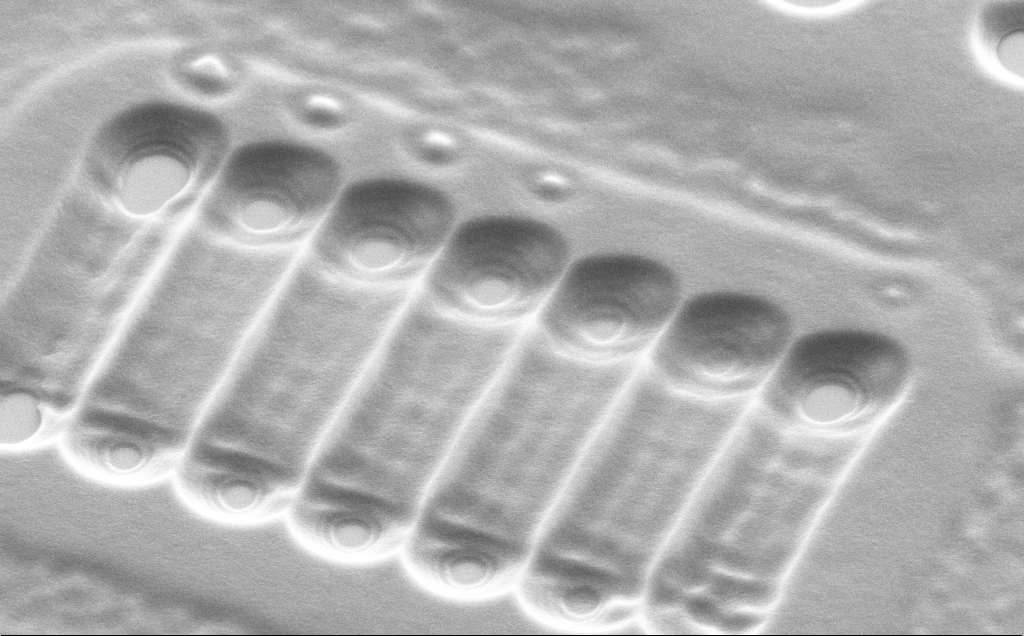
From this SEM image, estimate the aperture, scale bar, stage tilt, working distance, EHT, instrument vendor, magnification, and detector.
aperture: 30 µm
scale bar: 2000 nm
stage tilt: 45°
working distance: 10 mm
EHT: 5 kV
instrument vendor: Zeiss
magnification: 14.32 K X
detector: SE2